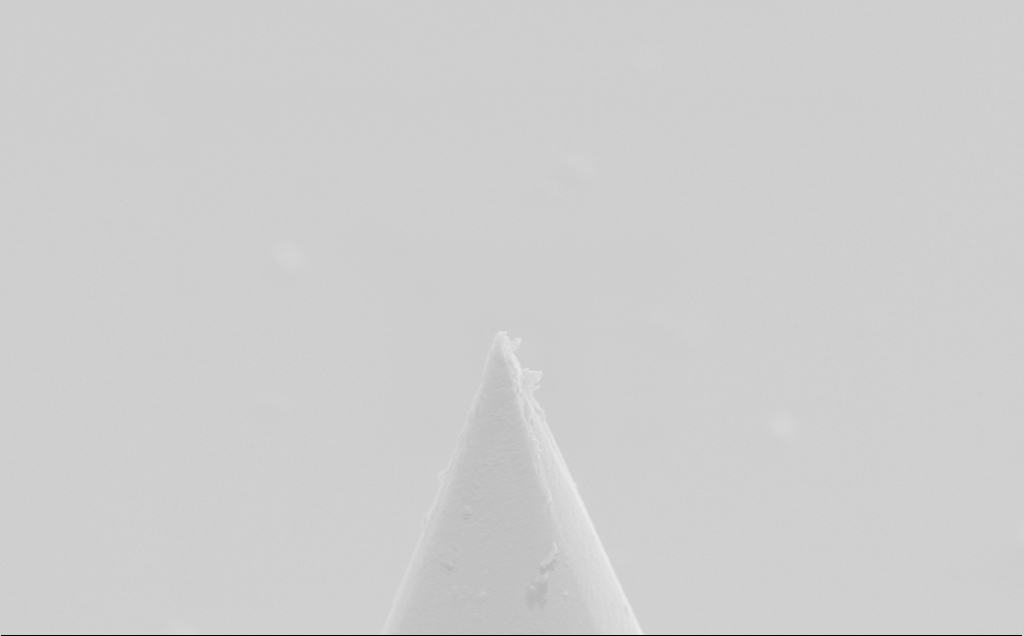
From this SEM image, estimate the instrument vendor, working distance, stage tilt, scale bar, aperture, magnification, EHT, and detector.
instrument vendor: Zeiss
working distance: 10 mm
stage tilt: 50°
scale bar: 1000 nm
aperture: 30 µm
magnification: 27.27 K X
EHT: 5 kV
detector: SE2